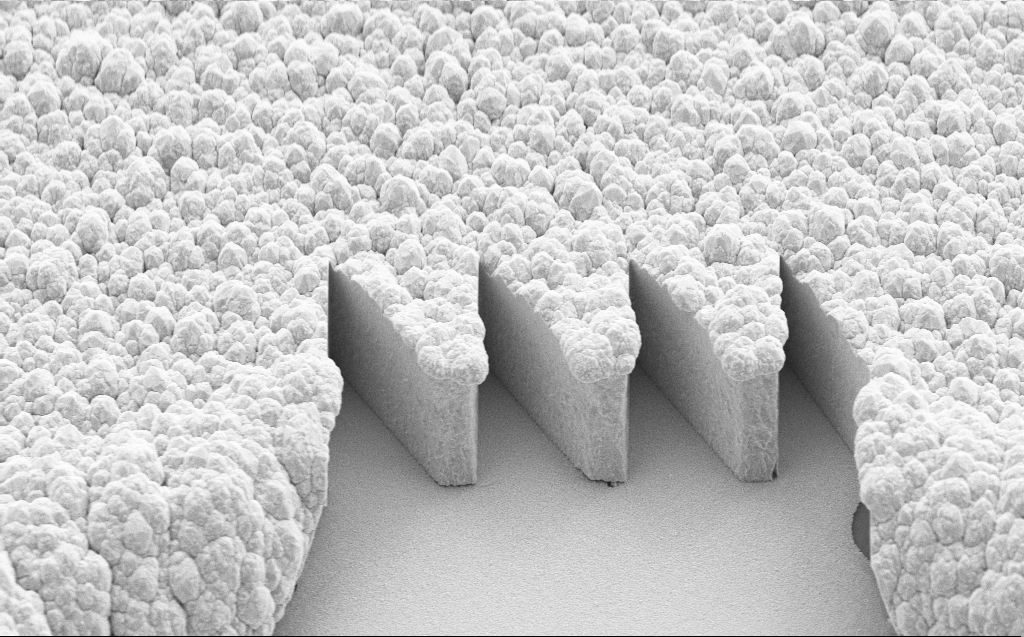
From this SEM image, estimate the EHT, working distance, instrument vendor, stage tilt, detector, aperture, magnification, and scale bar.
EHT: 10 kV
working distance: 7 mm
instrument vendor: Zeiss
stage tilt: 44.5°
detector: SE2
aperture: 30 µm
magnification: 4.8 K X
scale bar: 10000 nm